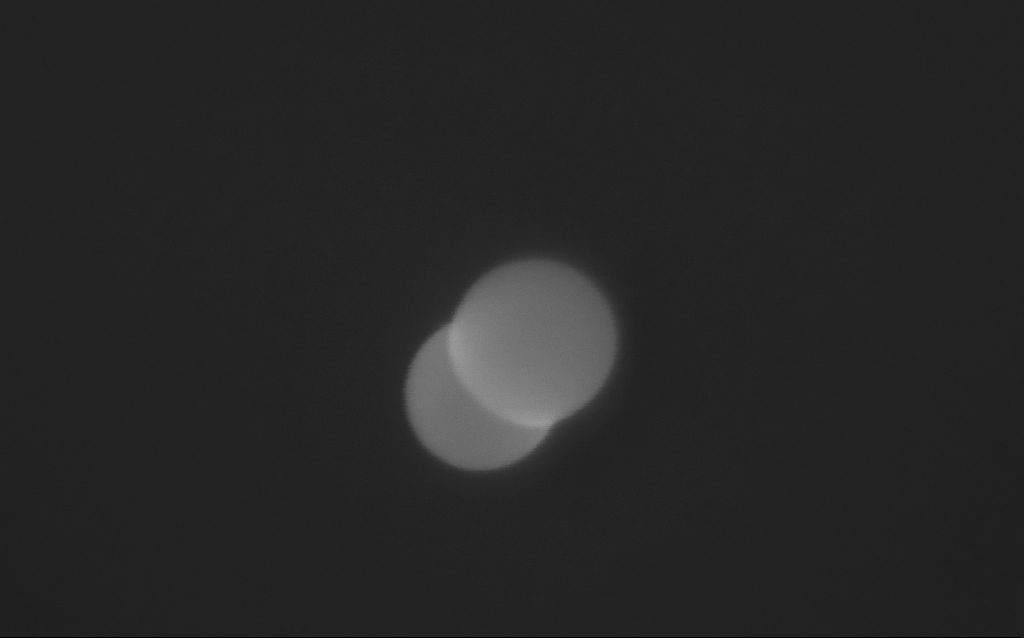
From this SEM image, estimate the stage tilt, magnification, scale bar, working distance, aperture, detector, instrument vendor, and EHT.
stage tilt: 22°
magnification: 457.01 K X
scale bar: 100 nm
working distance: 6 mm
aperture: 30 µm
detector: InLens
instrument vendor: Zeiss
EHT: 6 kV